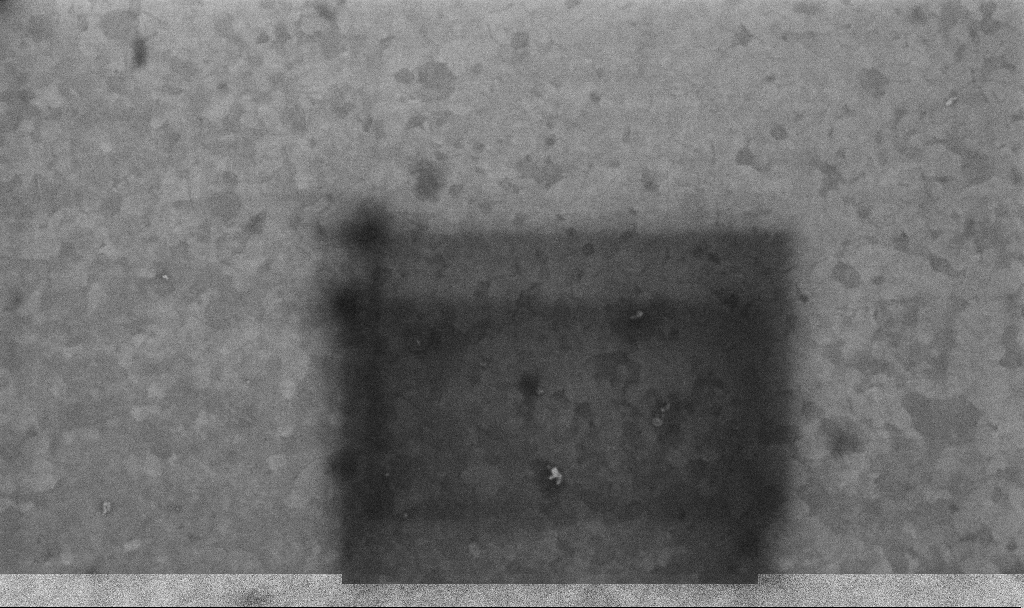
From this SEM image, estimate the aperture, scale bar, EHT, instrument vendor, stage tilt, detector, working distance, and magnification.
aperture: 30 µm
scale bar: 200 nm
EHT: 10 kV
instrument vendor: Zeiss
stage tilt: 0°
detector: InLens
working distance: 3.4 mm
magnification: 99.94 K X